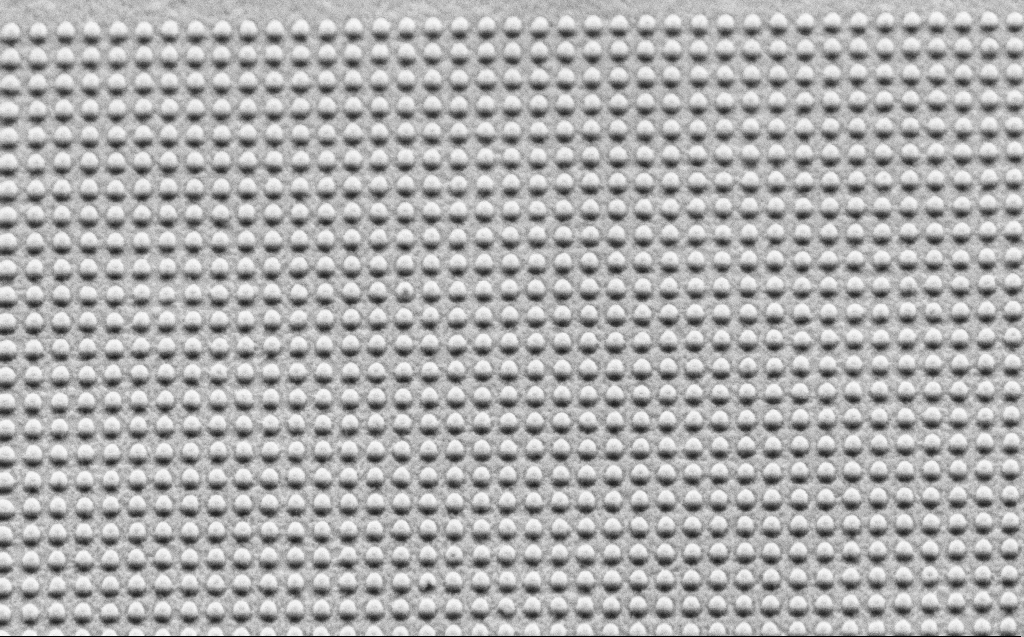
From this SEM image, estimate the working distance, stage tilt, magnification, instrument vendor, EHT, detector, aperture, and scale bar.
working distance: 6 mm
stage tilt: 44.1°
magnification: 24.46 K X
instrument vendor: Zeiss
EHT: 2.5 kV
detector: SE2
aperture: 30 µm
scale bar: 1000 nm